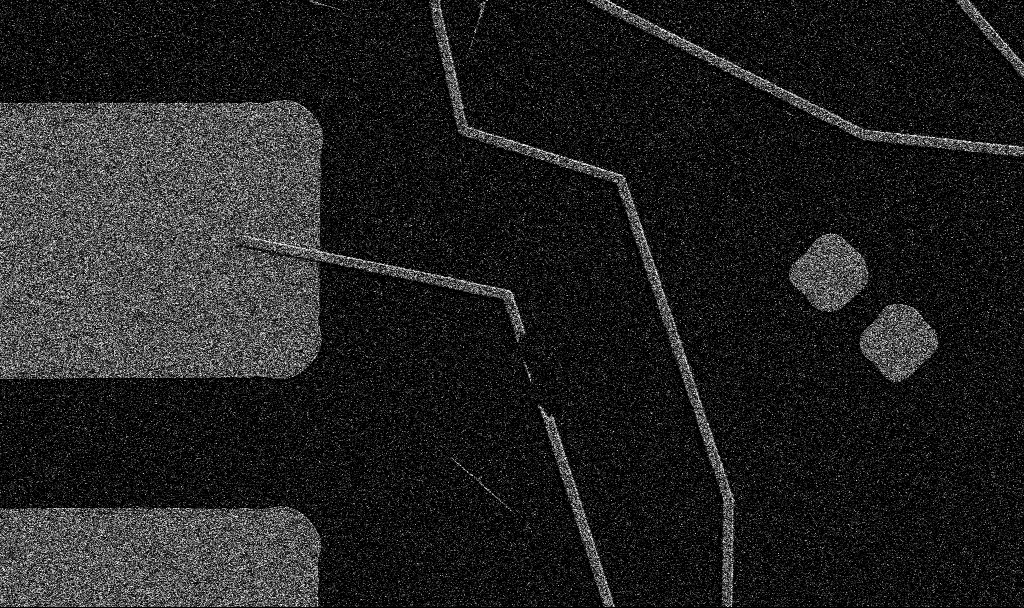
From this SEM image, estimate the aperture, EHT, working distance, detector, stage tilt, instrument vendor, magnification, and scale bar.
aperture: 30 µm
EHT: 5 kV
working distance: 10.7 mm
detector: SE2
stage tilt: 0°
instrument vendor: Zeiss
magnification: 5 K X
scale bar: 10000 nm